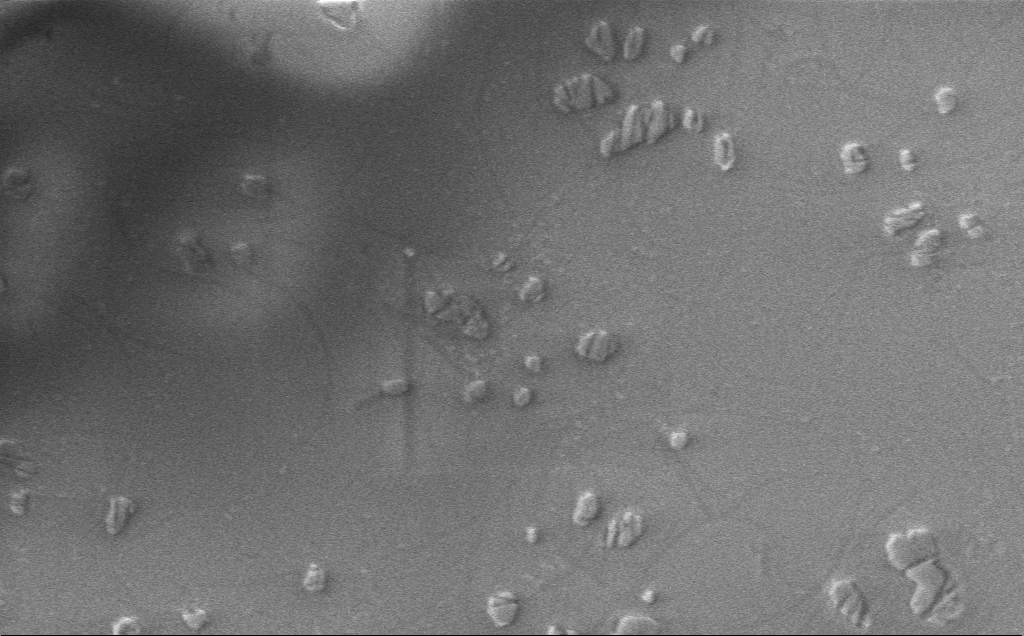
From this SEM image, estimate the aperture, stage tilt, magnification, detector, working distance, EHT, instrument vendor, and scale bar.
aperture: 30 µm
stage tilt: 0°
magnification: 10.03 K X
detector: InLens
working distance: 4 mm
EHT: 1 kV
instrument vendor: Zeiss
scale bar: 2000 nm